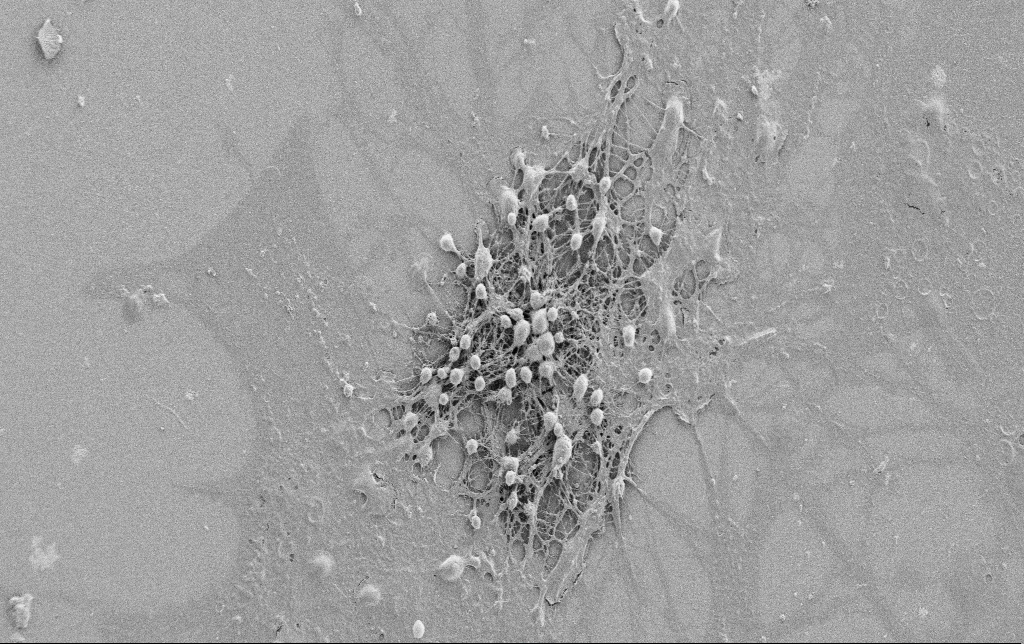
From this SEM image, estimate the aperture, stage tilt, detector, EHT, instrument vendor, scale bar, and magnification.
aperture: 30 µm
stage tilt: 0°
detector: SE2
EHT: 0.9 kV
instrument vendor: Zeiss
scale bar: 20000 nm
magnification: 1 K X